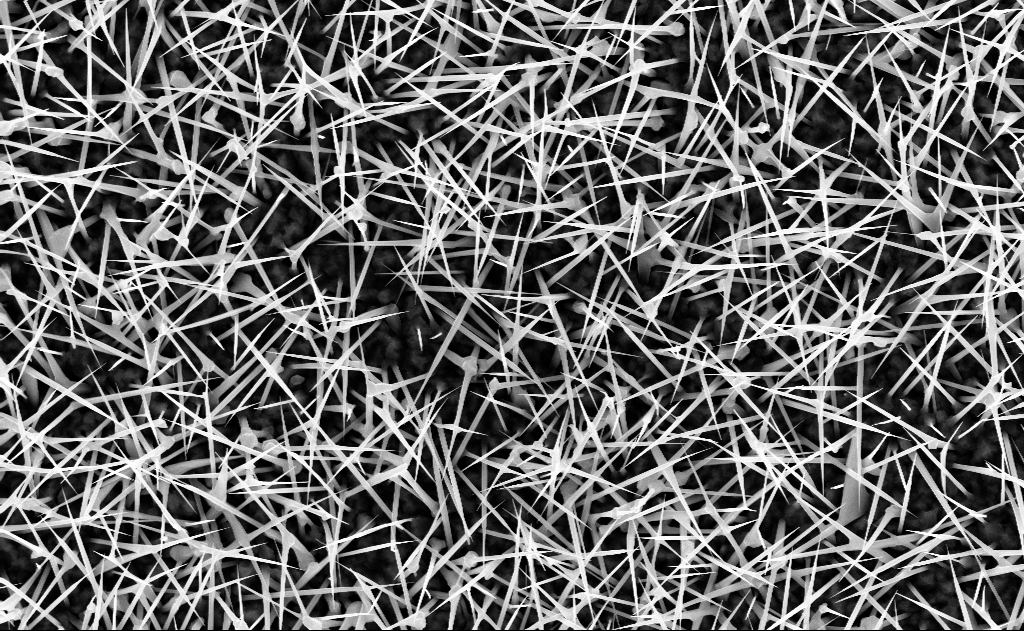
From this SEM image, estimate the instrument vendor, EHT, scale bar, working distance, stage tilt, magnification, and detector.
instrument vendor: Zeiss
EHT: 10 kV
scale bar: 2000 nm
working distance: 9 mm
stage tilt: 0°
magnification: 20 K X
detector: InLens